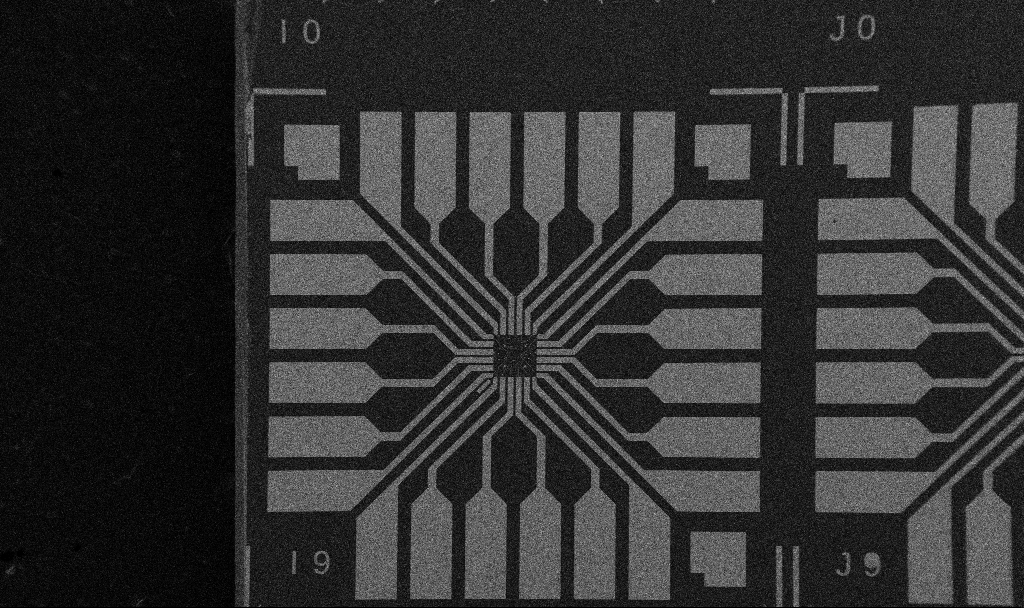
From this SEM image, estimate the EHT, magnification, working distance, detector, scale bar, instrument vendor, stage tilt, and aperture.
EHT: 5 kV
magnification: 0.1 K X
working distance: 10.7 mm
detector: SE2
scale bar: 200000 nm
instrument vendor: Zeiss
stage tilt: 0°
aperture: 30 µm